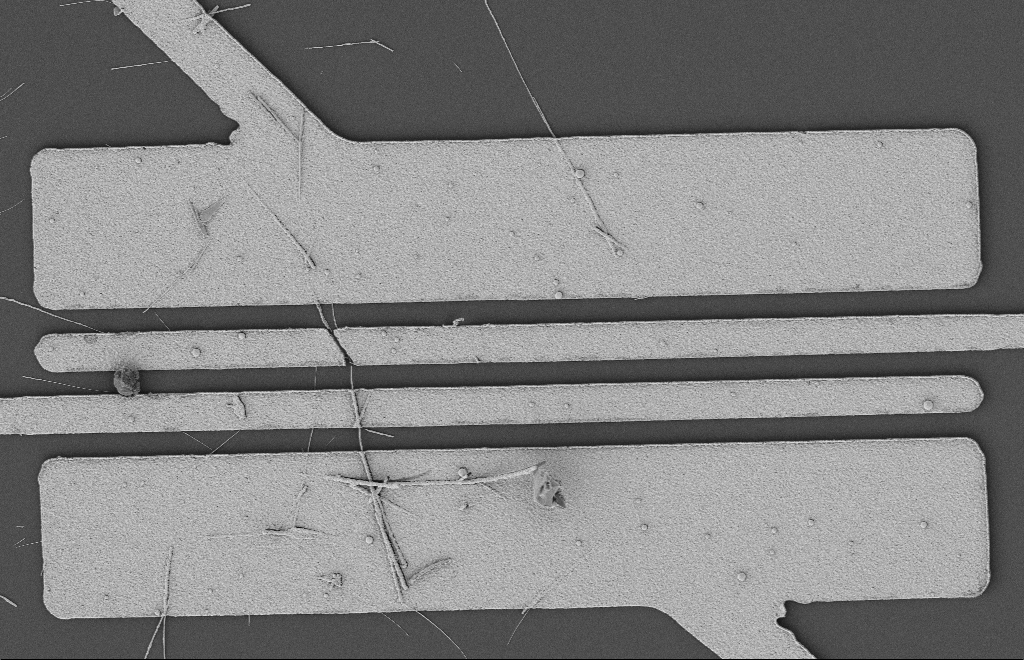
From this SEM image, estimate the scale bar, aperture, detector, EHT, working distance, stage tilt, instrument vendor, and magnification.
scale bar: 2000 nm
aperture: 20 µm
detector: SE2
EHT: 2 kV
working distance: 12 mm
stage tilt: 0°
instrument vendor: Zeiss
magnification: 5.65 K X